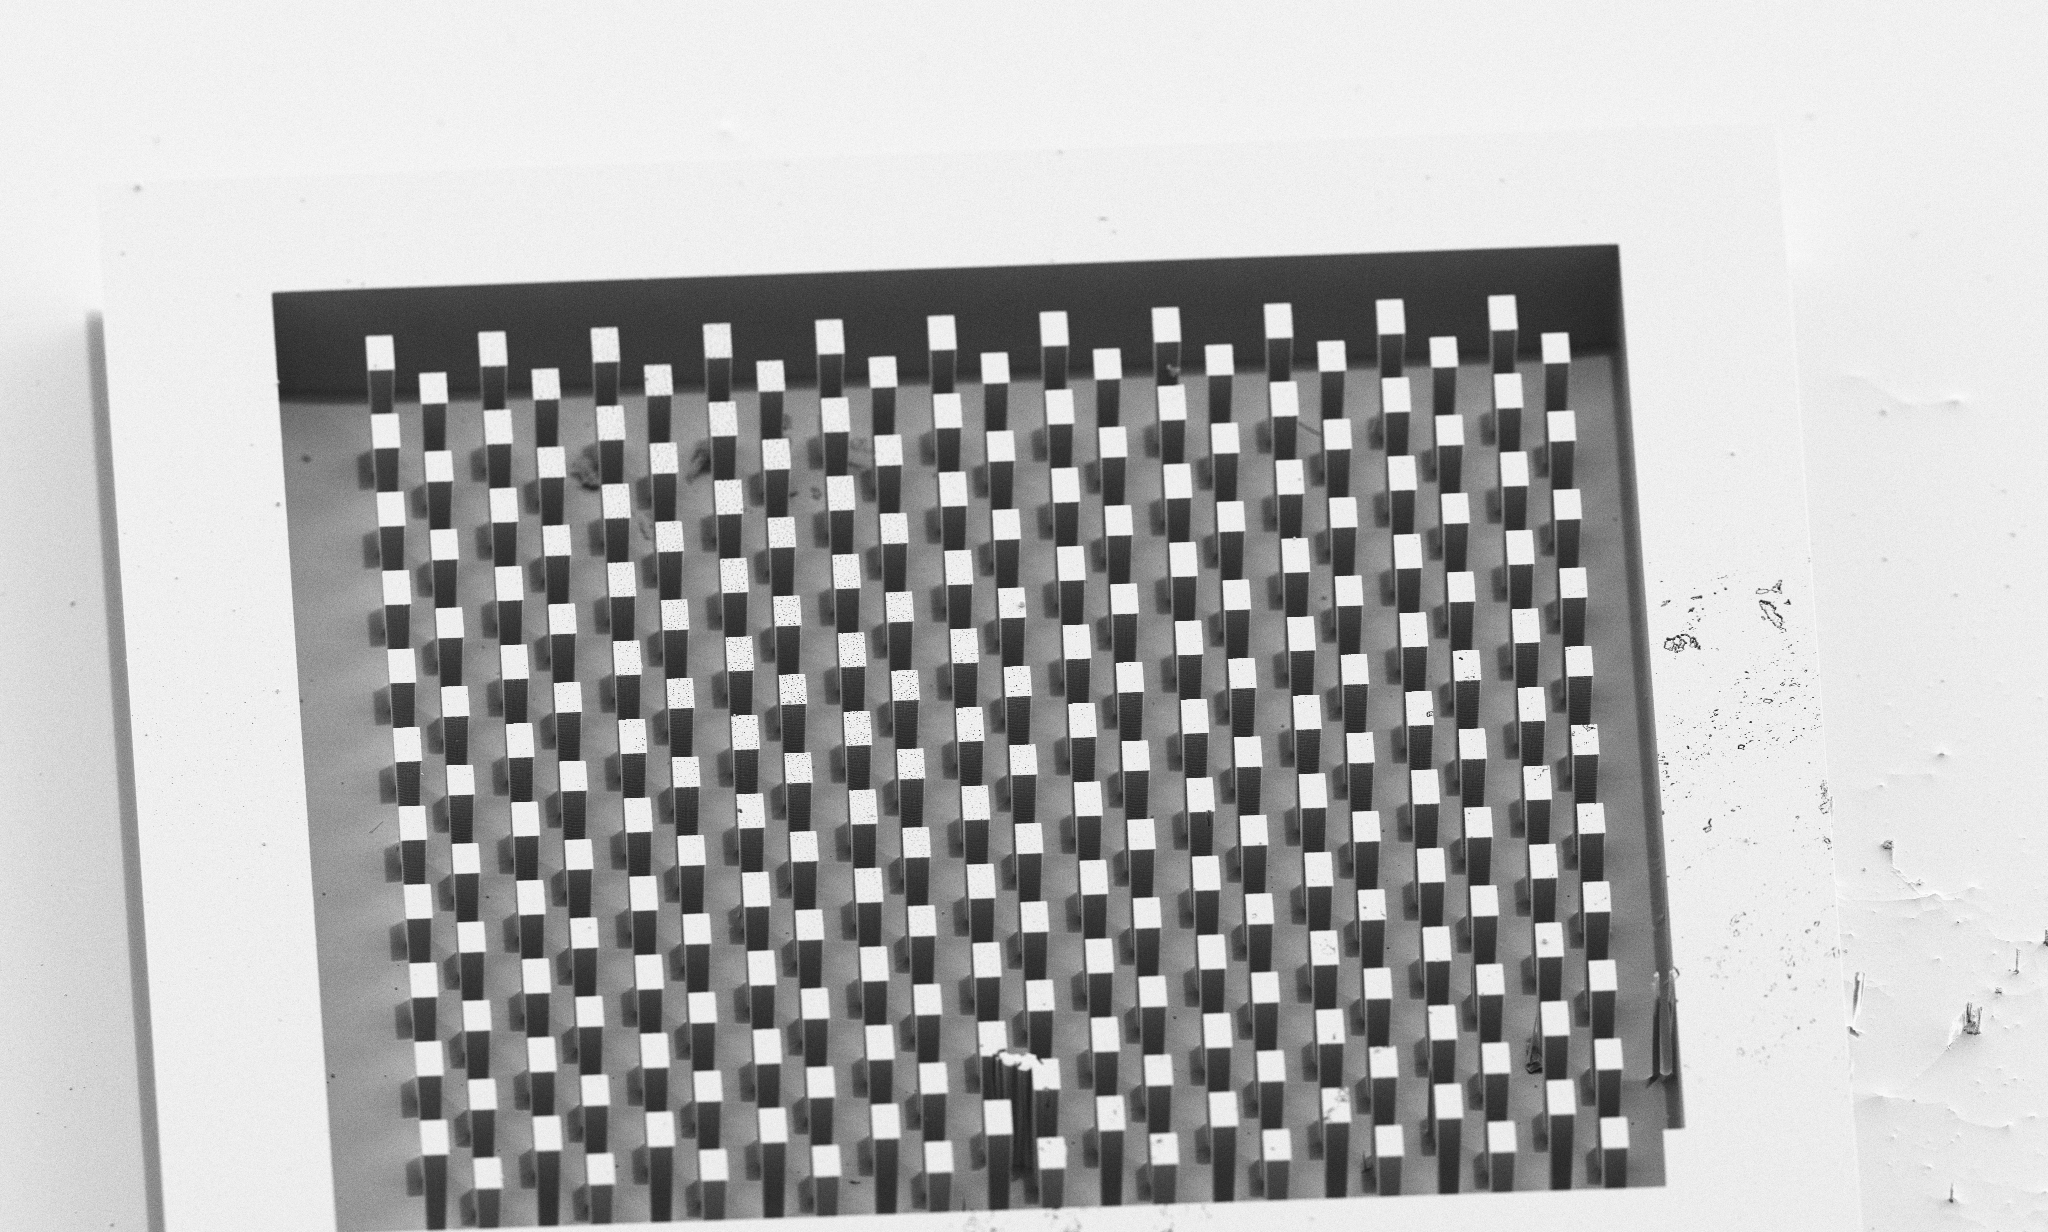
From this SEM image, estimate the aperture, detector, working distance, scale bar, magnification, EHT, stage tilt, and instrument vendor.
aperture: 30 µm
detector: SE2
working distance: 8 mm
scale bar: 20000 nm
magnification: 1.03 K X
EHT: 5 kV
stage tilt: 45°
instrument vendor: Zeiss